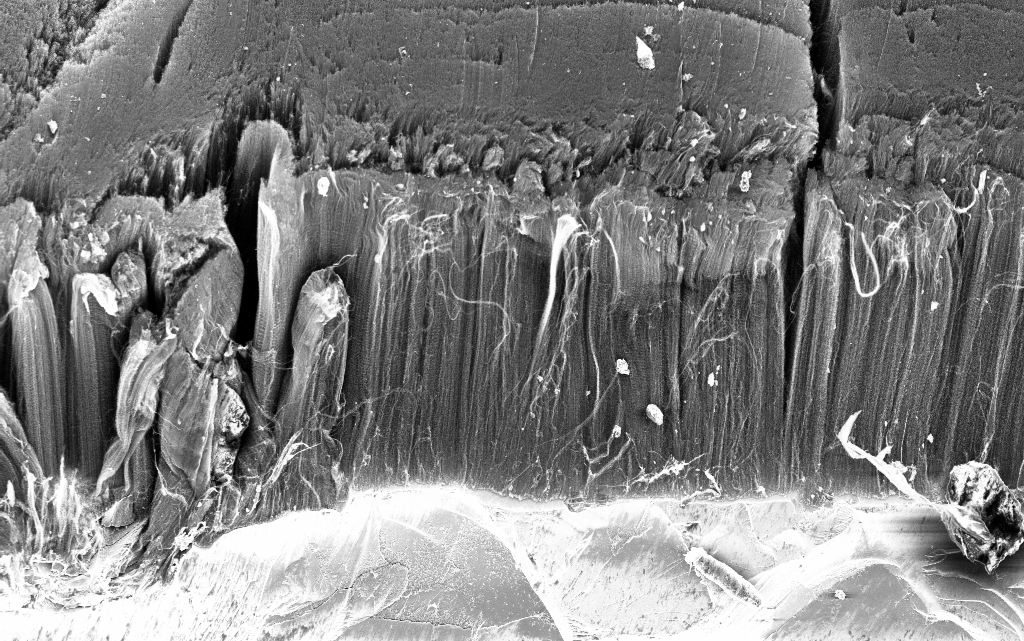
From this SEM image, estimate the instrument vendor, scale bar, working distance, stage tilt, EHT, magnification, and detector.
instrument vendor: Zeiss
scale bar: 20000 nm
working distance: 3.4 mm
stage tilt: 45°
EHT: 5 kV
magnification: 1 K X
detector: InLens